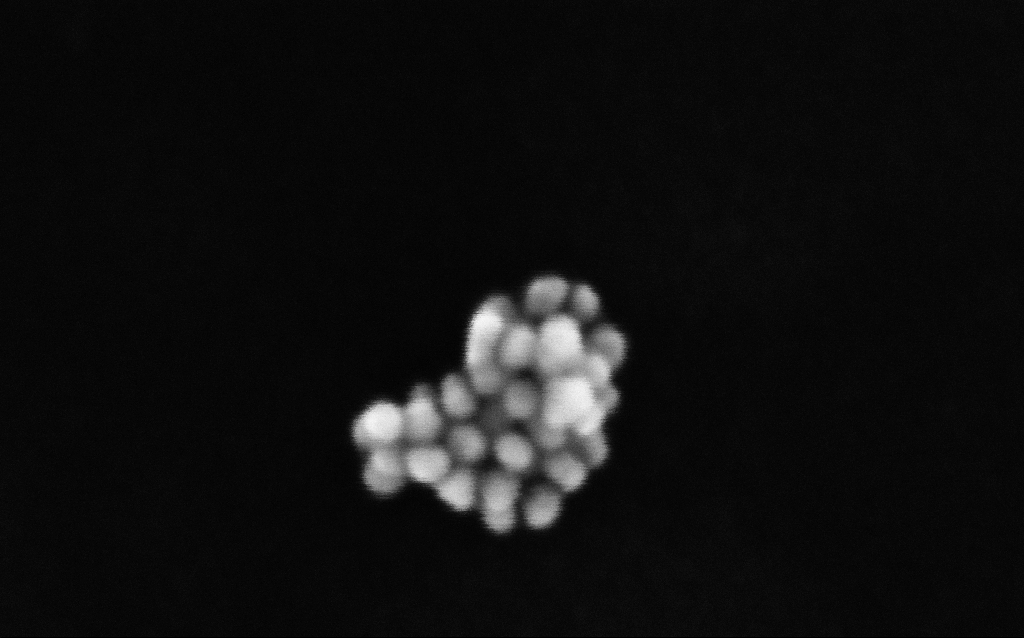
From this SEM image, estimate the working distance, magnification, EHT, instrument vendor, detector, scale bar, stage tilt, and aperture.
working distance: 3 mm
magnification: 965.88 K X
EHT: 6 kV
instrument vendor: Zeiss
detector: InLens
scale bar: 20 nm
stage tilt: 0°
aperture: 30 µm